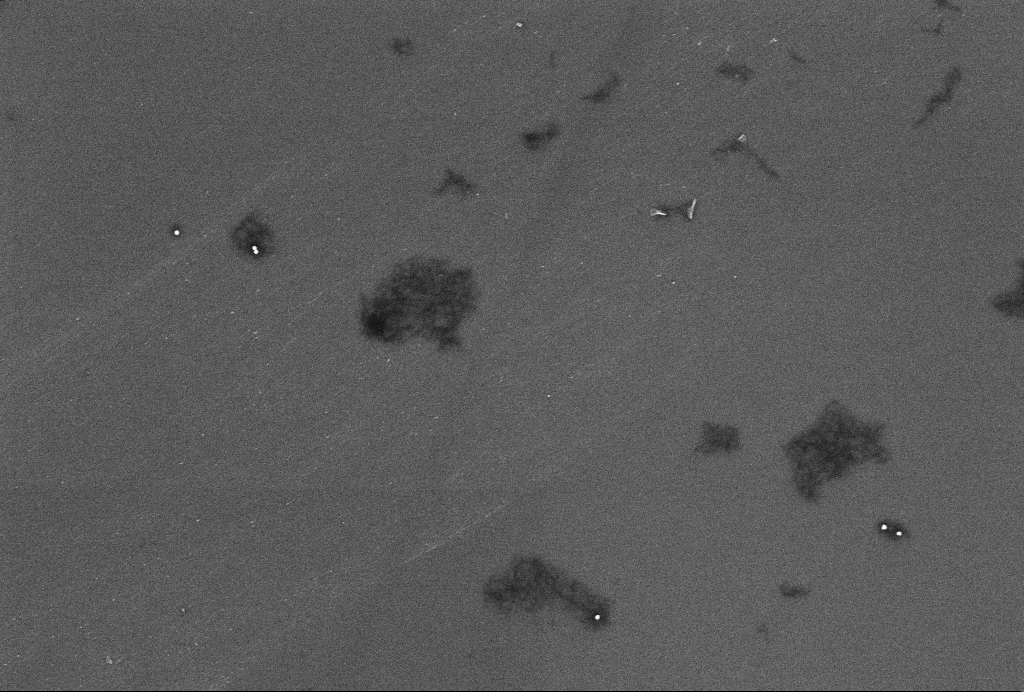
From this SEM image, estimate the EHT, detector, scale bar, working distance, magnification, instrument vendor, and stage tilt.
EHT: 2 kV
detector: InLens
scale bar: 200 nm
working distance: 3.3 mm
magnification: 61.65 K X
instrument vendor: Zeiss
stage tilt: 0°